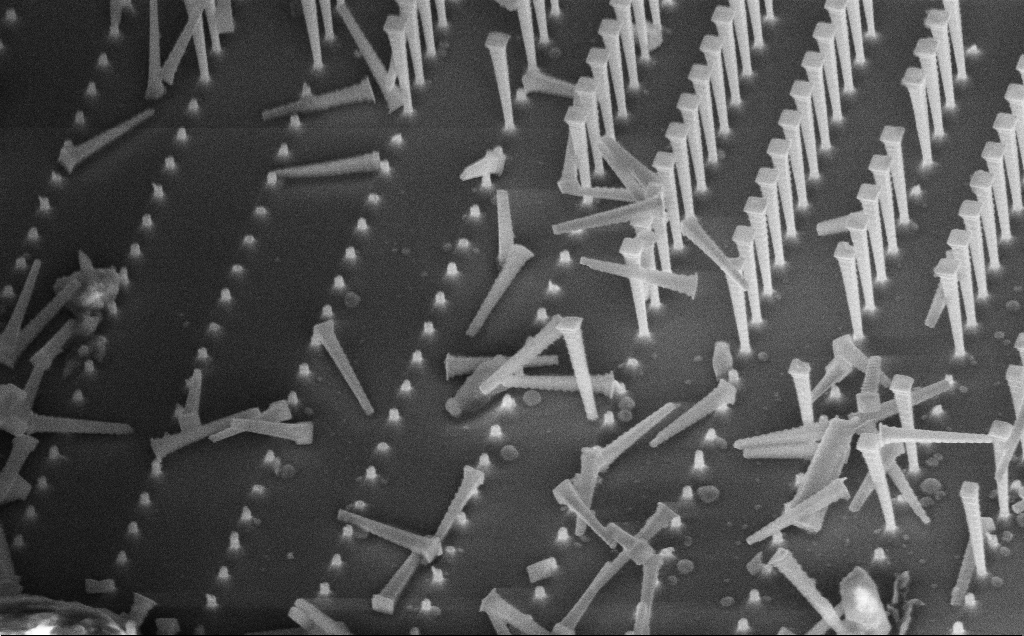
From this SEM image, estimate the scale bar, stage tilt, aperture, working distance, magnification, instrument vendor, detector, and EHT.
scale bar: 2000 nm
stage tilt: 45°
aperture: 30 µm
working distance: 8 mm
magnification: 7.81 K X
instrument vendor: Zeiss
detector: InLens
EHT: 10 kV